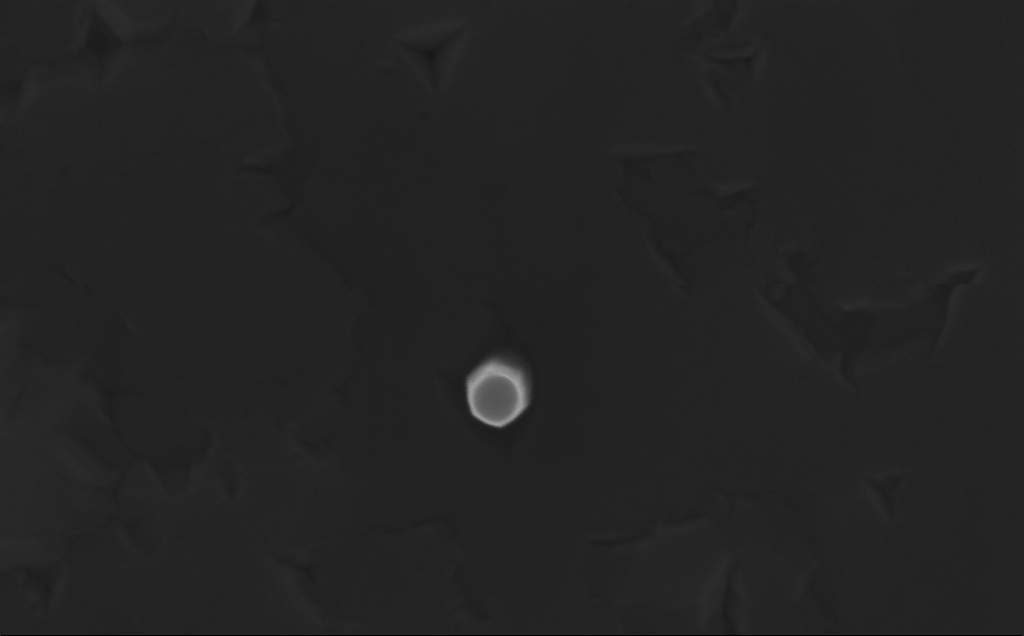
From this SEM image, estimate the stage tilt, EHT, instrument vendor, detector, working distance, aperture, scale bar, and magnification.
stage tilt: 0°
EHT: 10 kV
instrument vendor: Zeiss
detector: InLens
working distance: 6 mm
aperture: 30 µm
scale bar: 200 nm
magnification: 200 K X